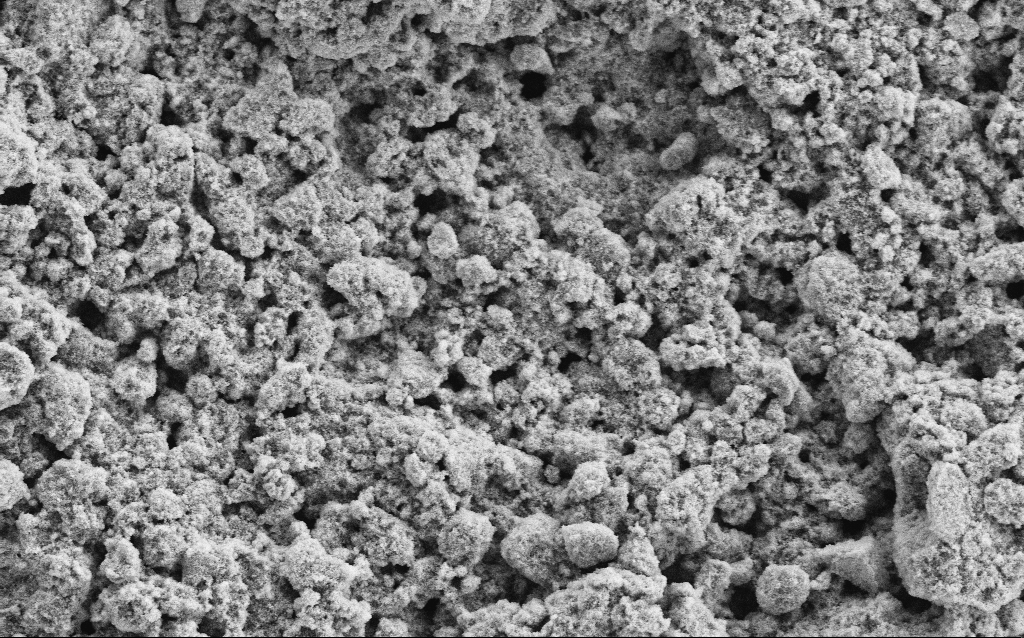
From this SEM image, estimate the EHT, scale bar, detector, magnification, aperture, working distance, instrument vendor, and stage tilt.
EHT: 5 kV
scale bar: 10000 nm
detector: SE2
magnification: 6.45 K X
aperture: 30 µm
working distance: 4.6 mm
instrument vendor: Zeiss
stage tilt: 0°